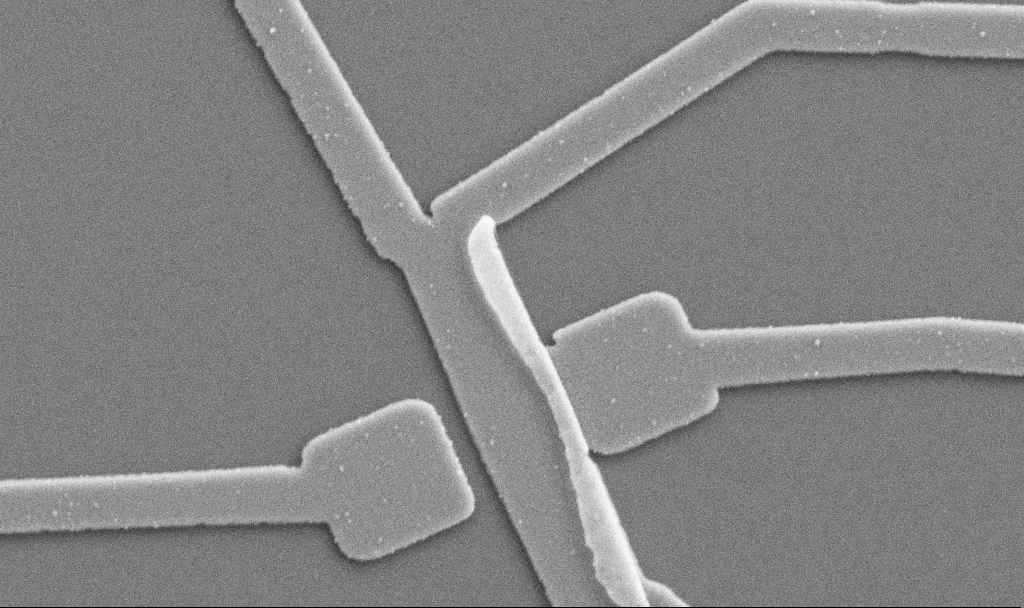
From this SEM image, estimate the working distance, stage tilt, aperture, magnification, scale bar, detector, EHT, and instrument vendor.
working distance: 10.7 mm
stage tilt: -0°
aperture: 30 µm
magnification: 20 K X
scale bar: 1000 nm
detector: SE2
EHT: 5 kV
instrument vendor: Zeiss